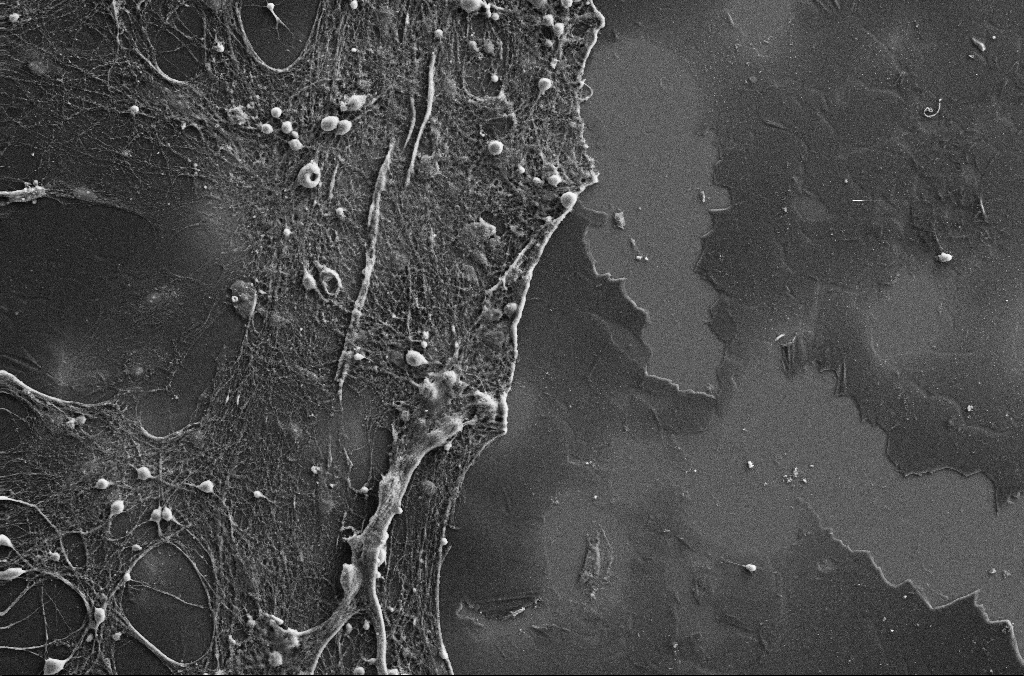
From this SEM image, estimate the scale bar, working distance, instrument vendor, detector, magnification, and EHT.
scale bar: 100000 nm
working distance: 3.1 mm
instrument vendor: Zeiss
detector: SE2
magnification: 0.5 K X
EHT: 15 kV